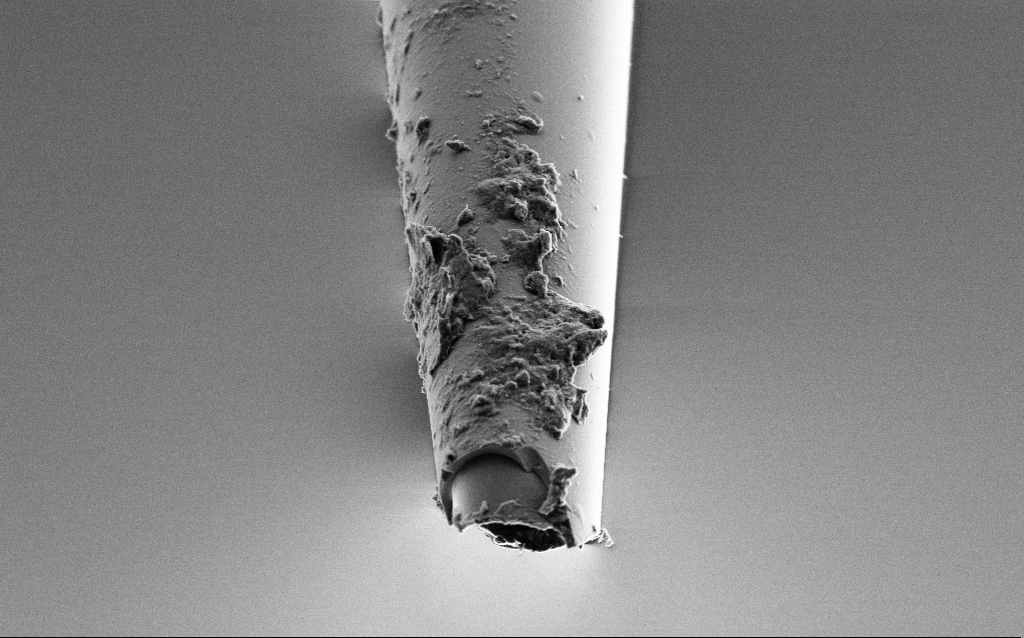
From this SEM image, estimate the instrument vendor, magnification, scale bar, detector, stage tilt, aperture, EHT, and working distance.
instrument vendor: Zeiss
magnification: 10 K X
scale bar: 2000 nm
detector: SE2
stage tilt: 45°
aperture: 30 µm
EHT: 2 kV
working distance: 6 mm